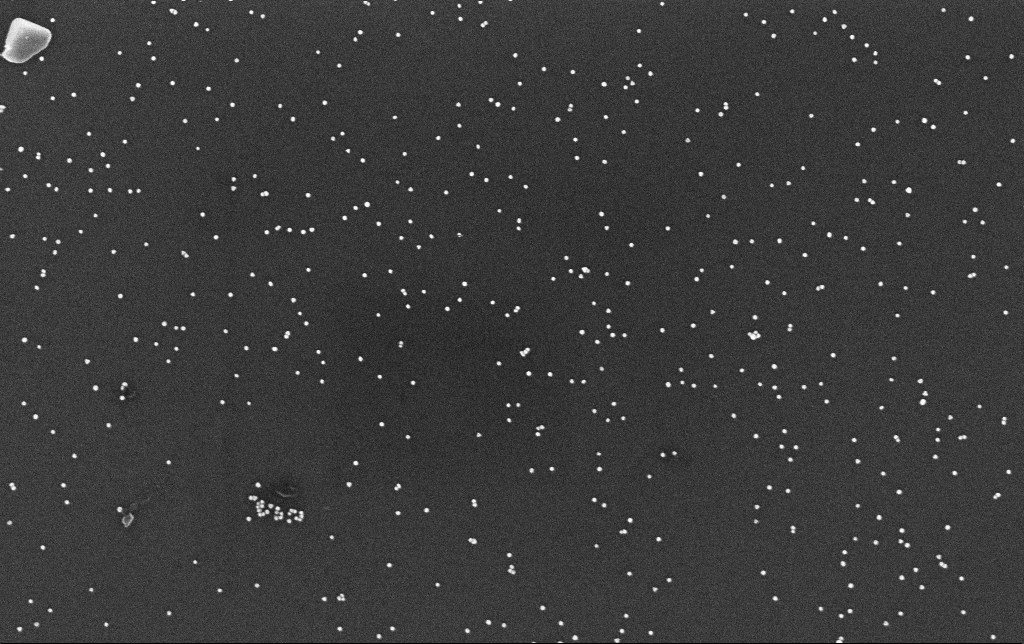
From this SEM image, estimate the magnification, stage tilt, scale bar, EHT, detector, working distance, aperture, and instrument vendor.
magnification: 100 K X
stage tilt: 0°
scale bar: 200 nm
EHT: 10 kV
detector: InLens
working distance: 3.4 mm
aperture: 30 µm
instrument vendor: Zeiss